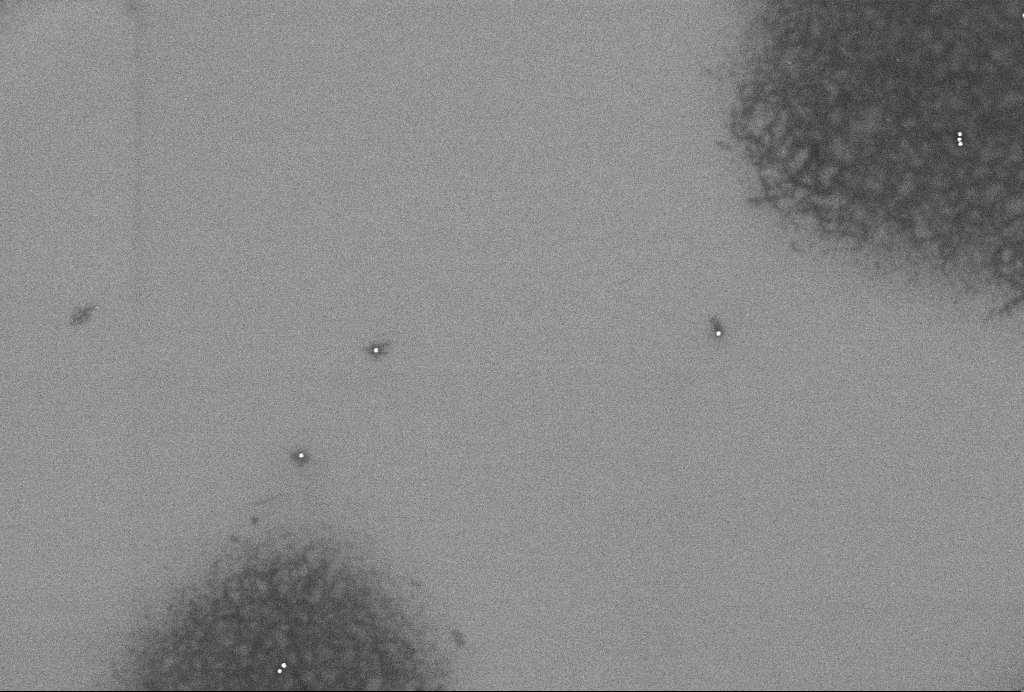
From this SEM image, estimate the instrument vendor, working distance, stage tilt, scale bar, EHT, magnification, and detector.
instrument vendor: Zeiss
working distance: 3.3 mm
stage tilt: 0°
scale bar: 1000 nm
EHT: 2 kV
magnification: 56.73 K X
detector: InLens